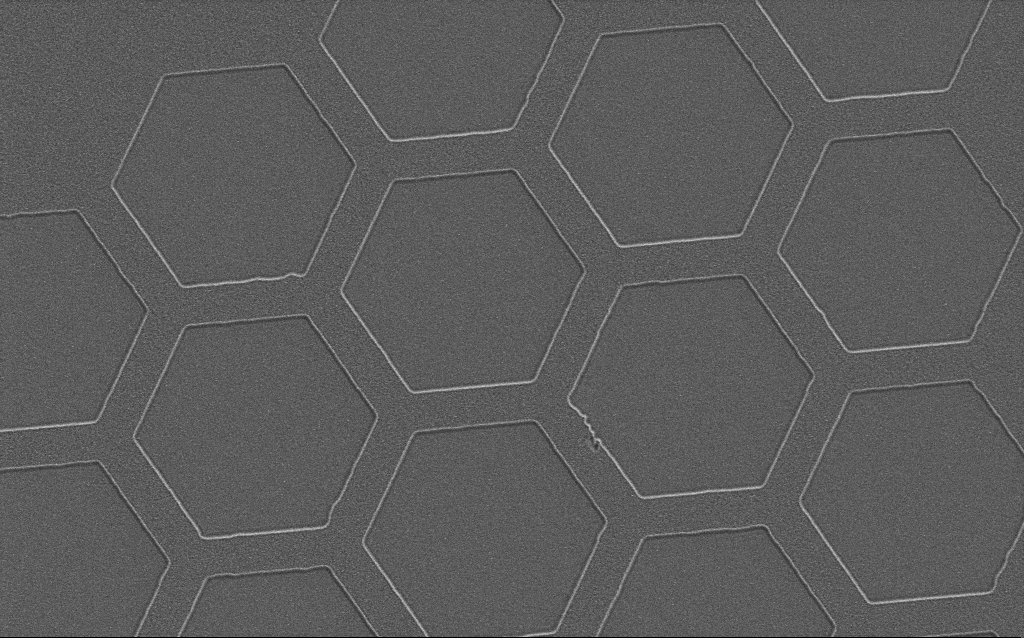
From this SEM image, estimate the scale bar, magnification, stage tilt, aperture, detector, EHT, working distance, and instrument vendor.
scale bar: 1000 nm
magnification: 14.99 K X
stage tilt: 0°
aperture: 30 µm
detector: SE2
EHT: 1.5 kV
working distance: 5 mm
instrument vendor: Zeiss